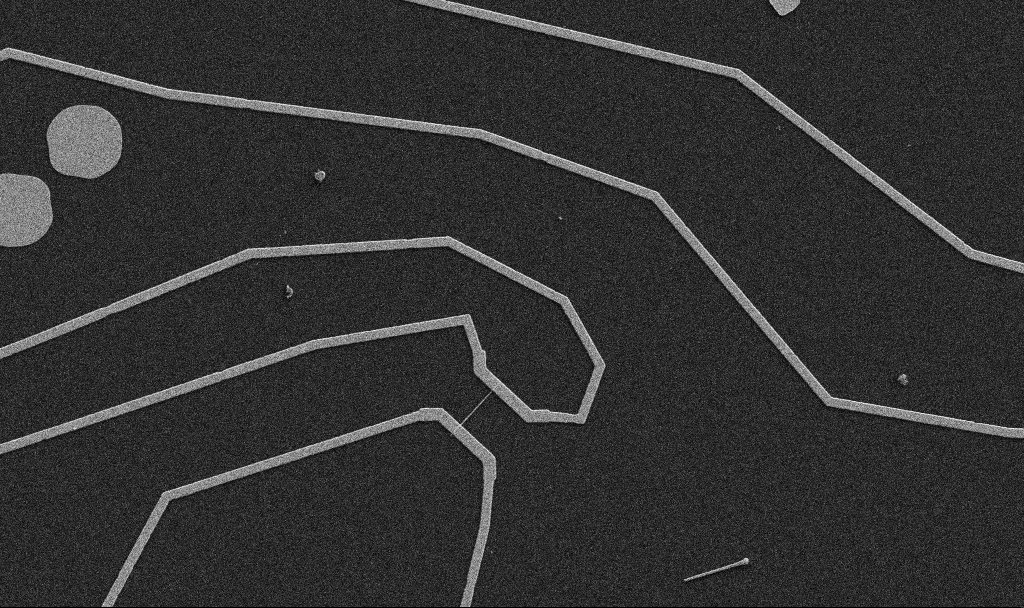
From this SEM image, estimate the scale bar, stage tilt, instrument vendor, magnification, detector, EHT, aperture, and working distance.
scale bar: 10000 nm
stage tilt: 0°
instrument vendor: Zeiss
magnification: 5 K X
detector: SE2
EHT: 5 kV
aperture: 30 µm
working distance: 10.7 mm